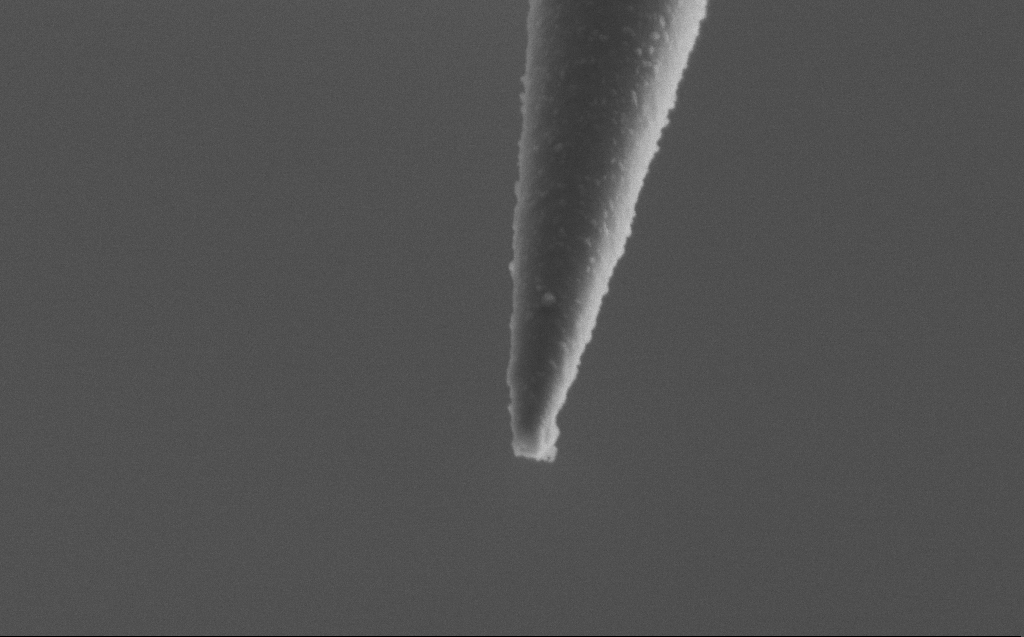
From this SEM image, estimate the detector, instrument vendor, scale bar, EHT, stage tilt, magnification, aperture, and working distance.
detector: SE2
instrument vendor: Zeiss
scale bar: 200 nm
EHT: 2 kV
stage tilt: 45°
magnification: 100 K X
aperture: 30 µm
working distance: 4 mm